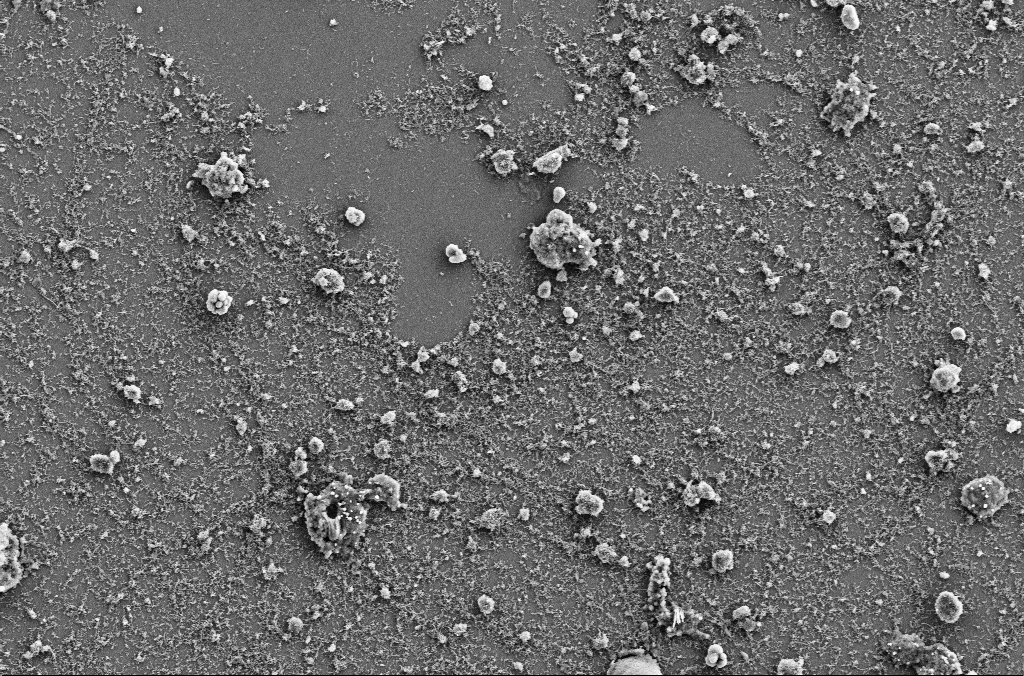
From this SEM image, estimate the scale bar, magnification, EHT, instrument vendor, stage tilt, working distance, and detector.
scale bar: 10000 nm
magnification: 4 K X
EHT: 5 kV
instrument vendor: Zeiss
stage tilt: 0°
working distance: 4 mm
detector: SE2